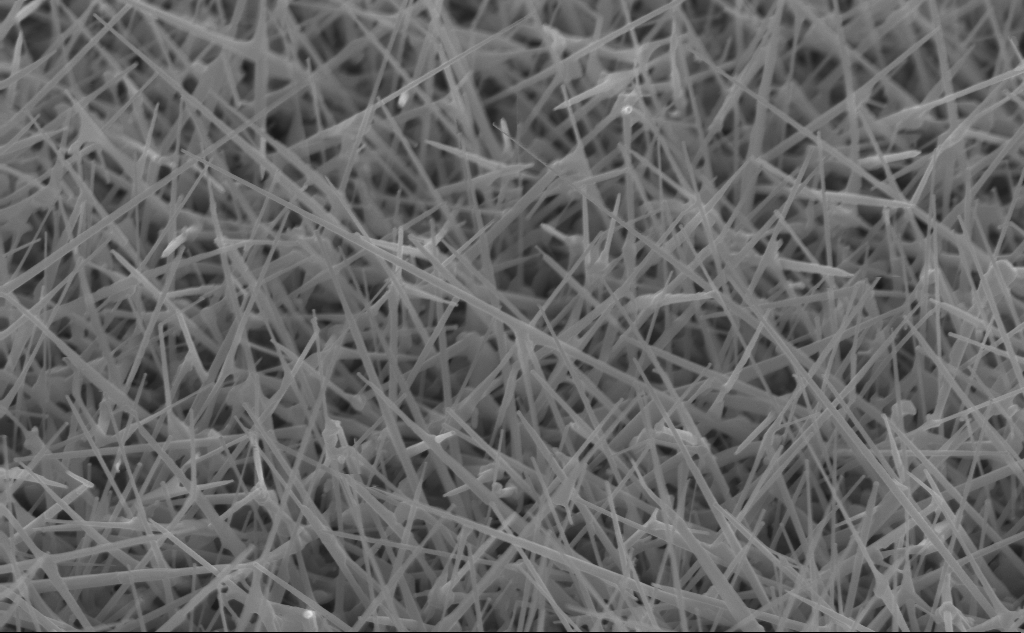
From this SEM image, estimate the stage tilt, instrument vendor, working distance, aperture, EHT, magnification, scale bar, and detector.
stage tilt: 45°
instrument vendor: Zeiss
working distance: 4 mm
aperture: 30 µm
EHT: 10 kV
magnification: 40 K X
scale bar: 1000 nm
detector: InLens